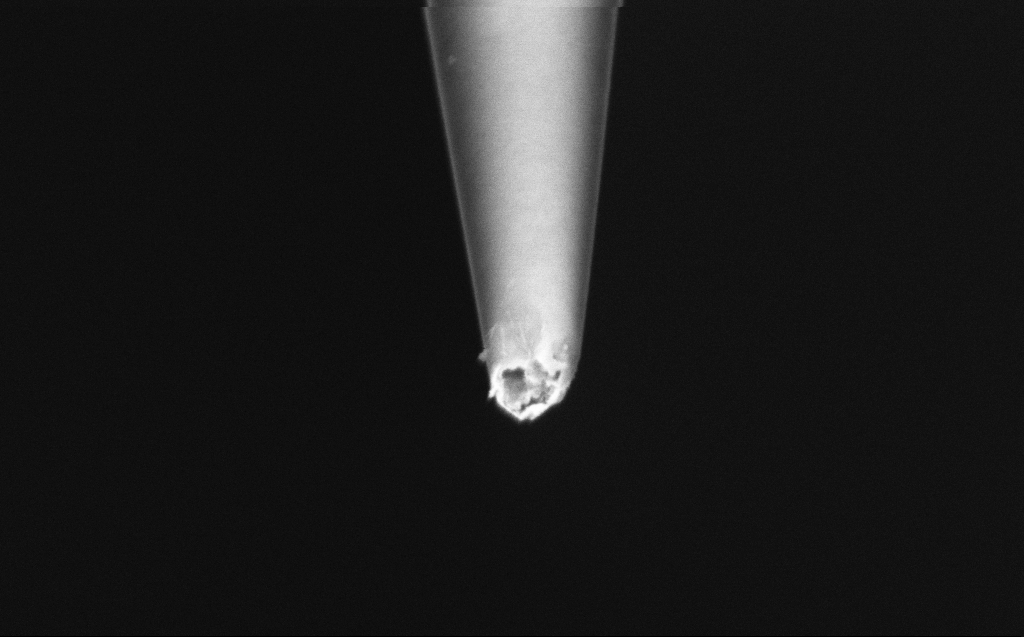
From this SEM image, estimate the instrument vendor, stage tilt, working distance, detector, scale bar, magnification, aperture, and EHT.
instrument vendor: Zeiss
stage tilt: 45°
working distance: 6 mm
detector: InLens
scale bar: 200 nm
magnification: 100 K X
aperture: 30 µm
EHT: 2 kV